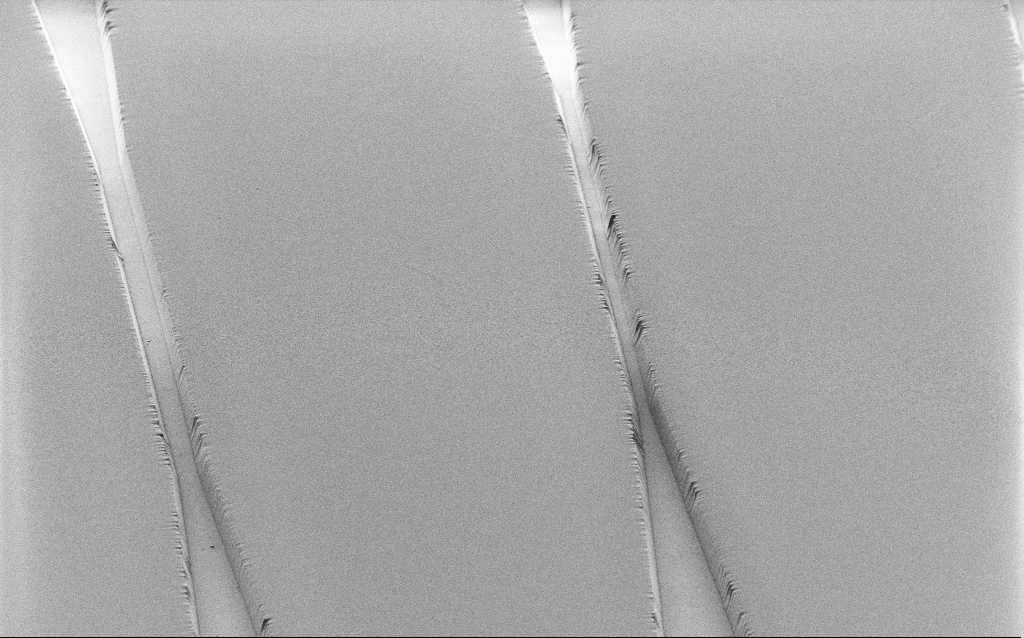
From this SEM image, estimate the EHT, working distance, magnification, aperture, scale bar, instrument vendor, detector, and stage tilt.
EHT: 1.2 kV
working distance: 8 mm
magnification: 1.63 K X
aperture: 30 µm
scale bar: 20000 nm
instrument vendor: Zeiss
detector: SE2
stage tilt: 45°